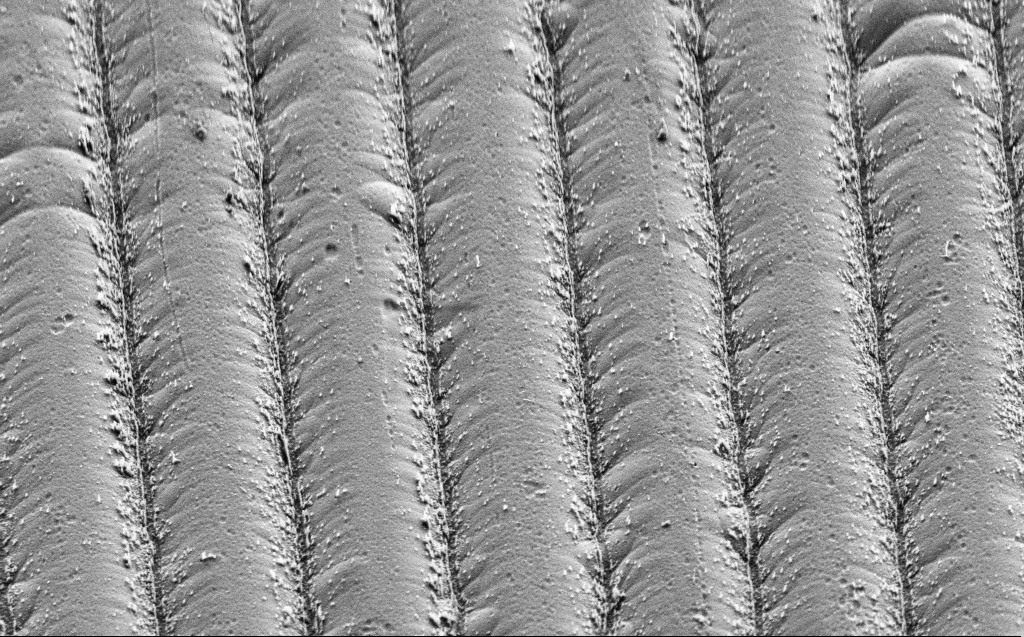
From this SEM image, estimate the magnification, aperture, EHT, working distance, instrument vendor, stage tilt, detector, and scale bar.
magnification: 5.46 K X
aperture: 30 µm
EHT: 3 kV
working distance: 9 mm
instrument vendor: Zeiss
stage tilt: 45°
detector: SE2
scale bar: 10000 nm